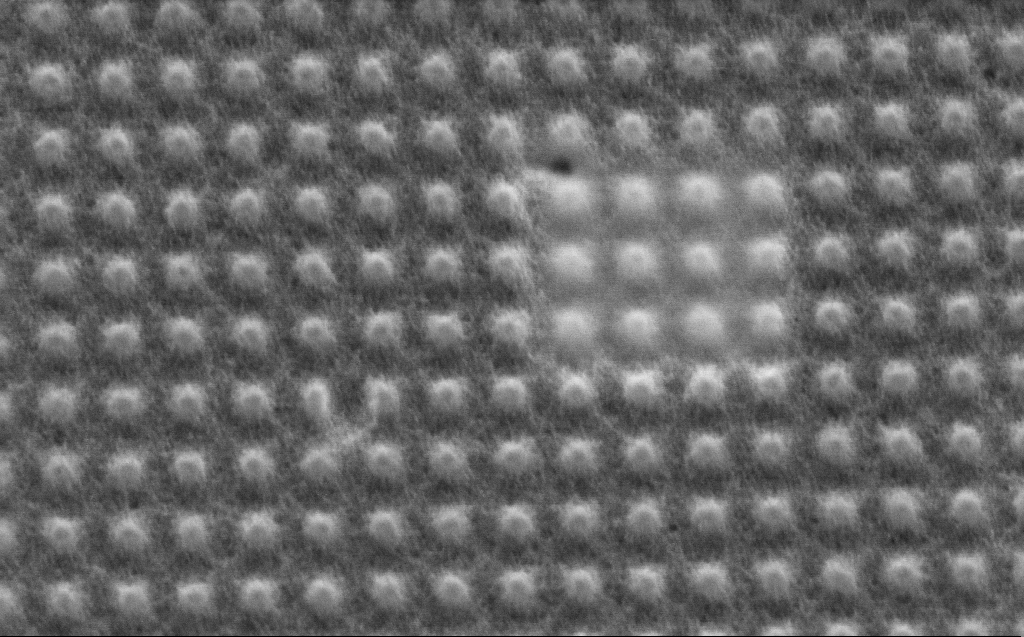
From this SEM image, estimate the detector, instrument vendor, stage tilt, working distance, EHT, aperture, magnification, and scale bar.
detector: InLens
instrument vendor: Zeiss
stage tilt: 29.8°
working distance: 4 mm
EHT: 2 kV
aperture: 30 µm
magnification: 120.7 K X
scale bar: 200 nm